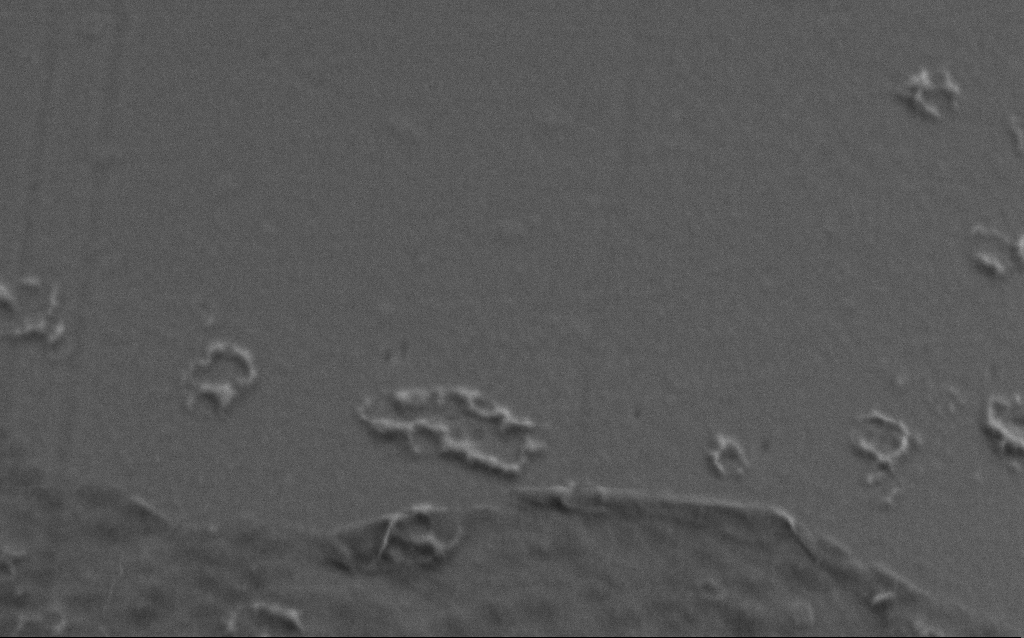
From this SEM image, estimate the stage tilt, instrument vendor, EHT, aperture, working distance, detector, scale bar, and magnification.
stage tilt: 45°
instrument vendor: Zeiss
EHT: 1 kV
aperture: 30 µm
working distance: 6 mm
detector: SE2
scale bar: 200 nm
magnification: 100 K X